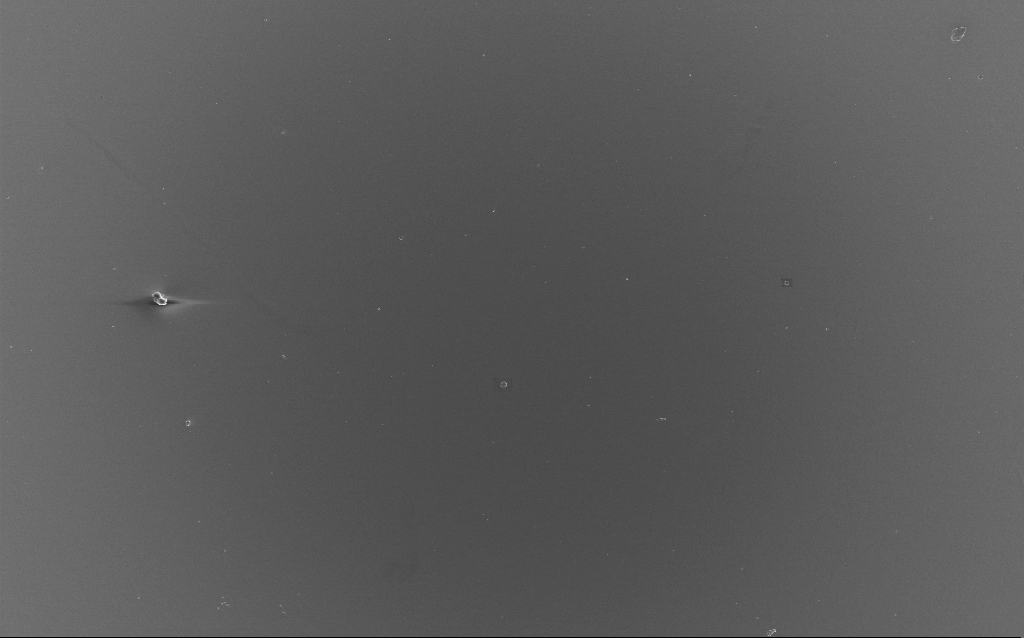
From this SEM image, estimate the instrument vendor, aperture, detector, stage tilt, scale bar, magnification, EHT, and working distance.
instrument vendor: Zeiss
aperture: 30 µm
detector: InLens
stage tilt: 0°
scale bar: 100000 nm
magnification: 0.64 K X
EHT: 10 kV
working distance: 2 mm